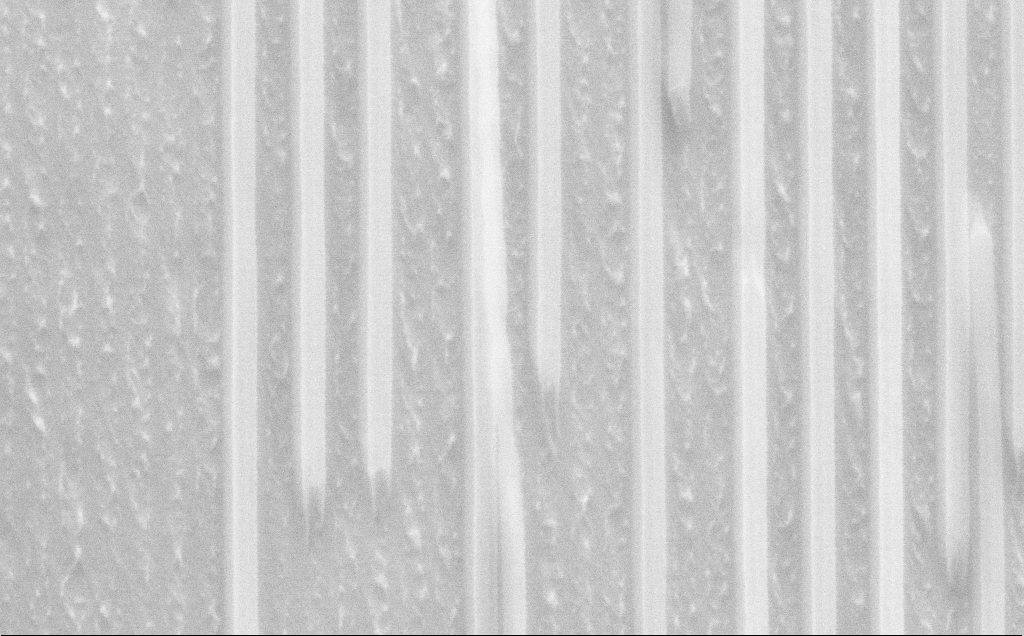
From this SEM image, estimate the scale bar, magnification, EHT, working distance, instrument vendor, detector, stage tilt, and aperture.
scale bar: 200 nm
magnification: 84.69 K X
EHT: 10 kV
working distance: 8 mm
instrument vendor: Zeiss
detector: SE2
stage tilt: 45°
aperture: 30 µm